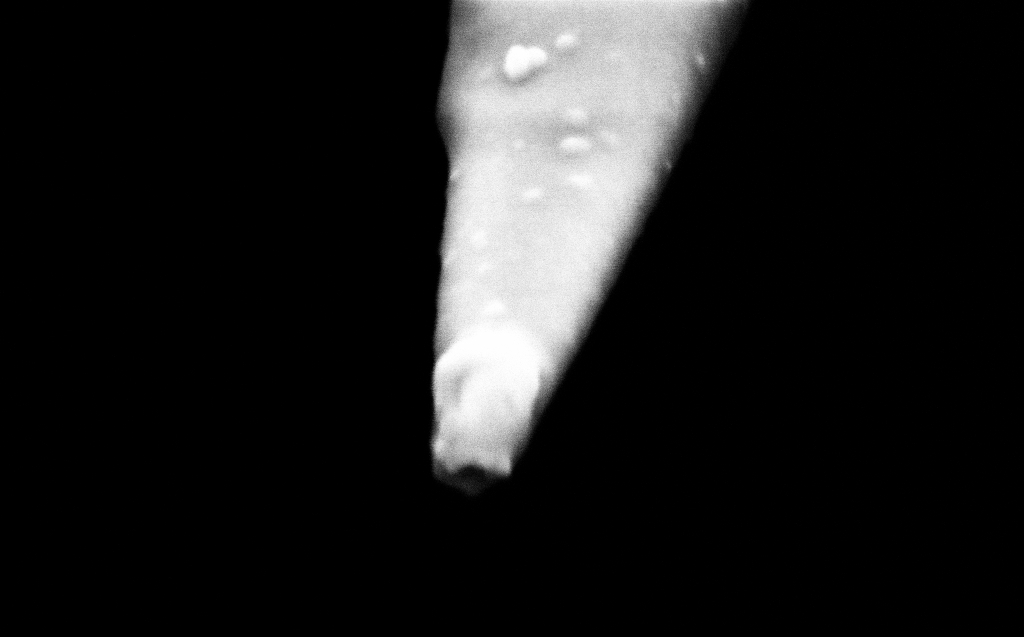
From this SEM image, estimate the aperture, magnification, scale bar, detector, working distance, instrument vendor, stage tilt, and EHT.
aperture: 30 µm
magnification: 250 K X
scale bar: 200 nm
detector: InLens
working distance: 5 mm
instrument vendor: Zeiss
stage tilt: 45°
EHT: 2 kV